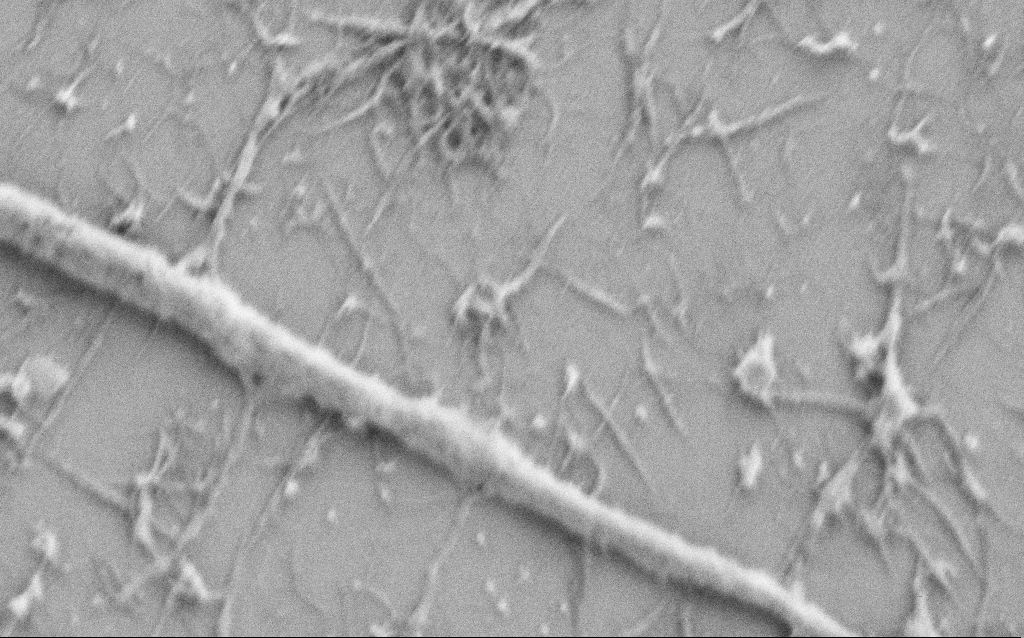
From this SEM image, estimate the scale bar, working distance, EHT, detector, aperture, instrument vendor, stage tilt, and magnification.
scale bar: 1000 nm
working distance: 6.8 mm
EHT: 1 kV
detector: SE2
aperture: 30 µm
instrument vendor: Zeiss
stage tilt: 0°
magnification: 50 K X